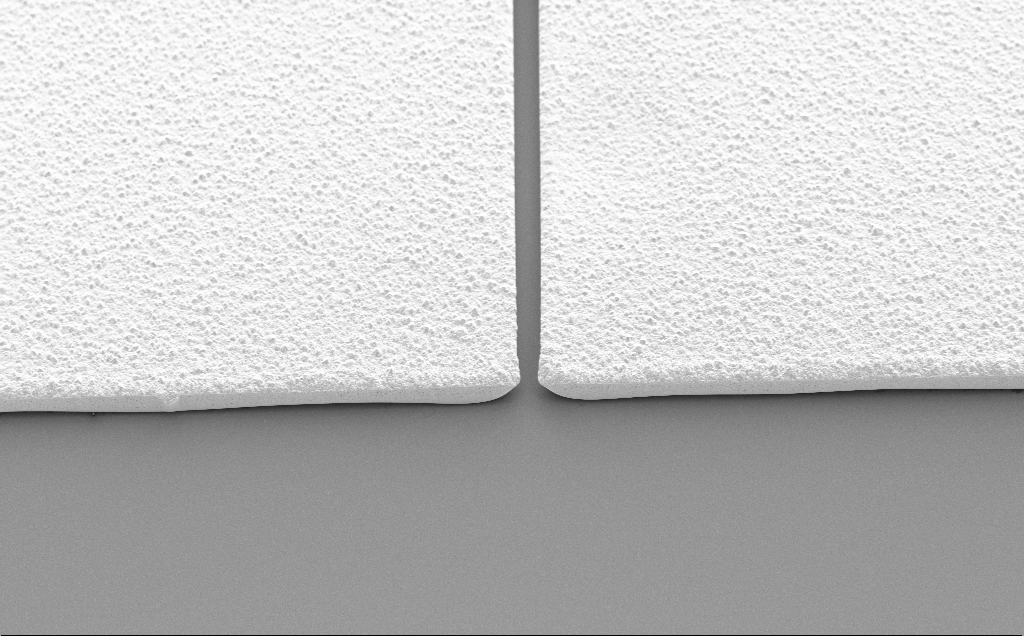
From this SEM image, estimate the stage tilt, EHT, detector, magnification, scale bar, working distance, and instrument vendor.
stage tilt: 45°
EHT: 5 kV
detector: SE2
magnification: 1.04 K X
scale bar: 20000 nm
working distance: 14 mm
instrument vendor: Zeiss